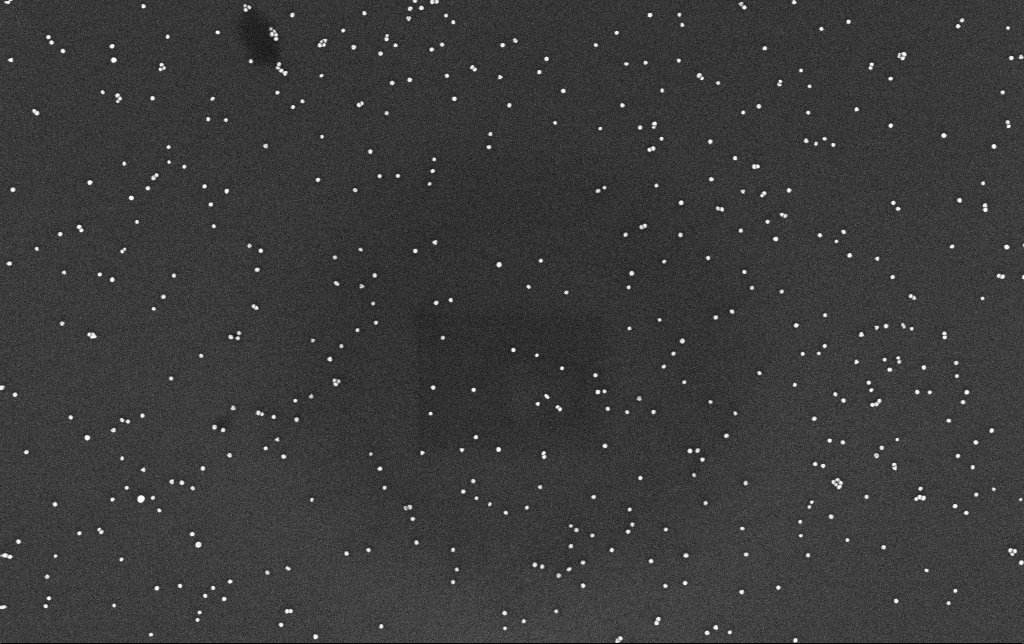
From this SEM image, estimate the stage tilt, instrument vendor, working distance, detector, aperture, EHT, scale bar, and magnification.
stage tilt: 0°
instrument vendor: Zeiss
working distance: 3.4 mm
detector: InLens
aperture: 30 µm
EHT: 10 kV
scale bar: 200 nm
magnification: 100 K X